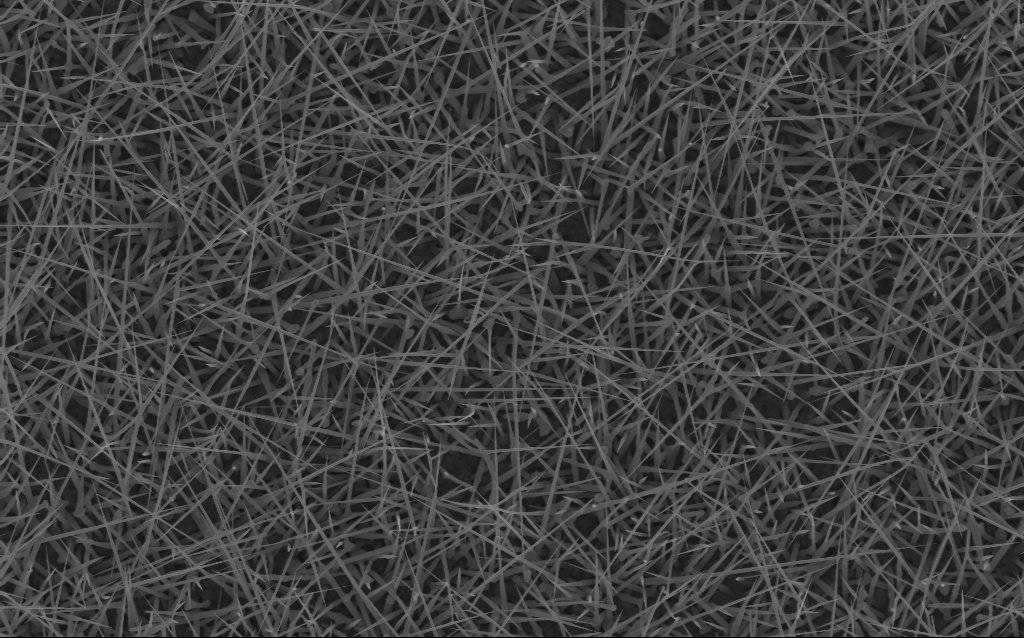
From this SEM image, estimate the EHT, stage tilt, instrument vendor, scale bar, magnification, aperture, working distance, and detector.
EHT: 10 kV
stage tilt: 0°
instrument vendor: Zeiss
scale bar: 2000 nm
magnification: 10 K X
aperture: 30 µm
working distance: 7 mm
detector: InLens